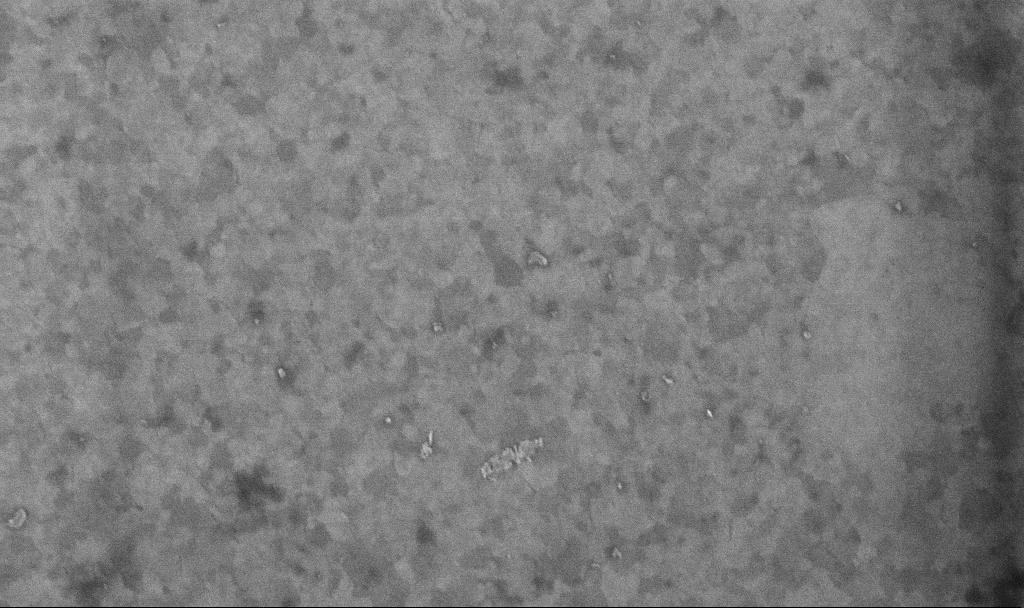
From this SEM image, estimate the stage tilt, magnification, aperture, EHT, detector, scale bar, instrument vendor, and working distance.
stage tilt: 0°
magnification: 100.94 K X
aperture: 30 µm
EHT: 10 kV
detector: InLens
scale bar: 200 nm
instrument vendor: Zeiss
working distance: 3.4 mm